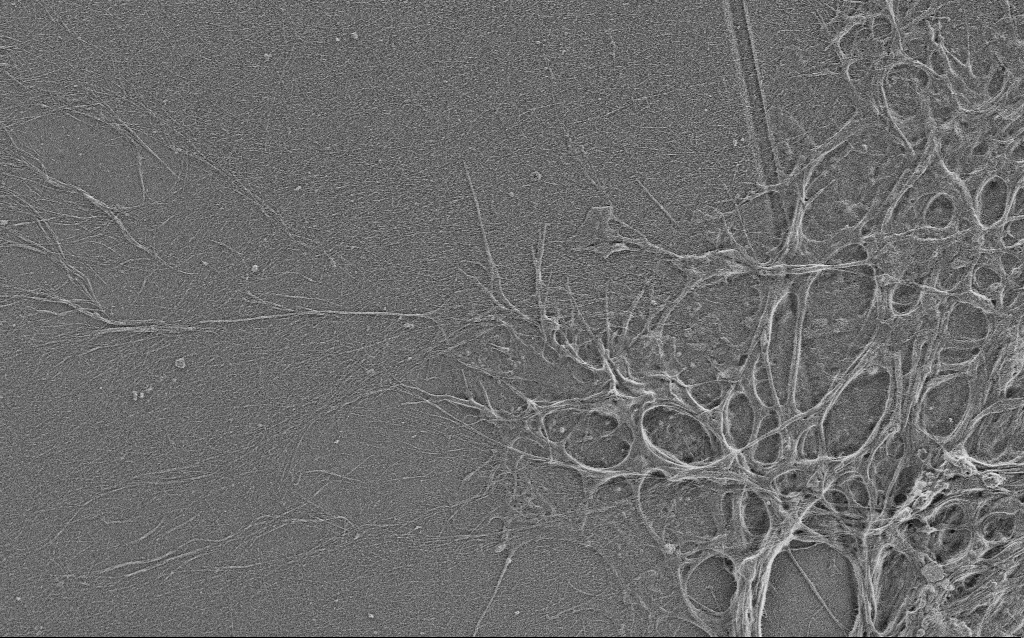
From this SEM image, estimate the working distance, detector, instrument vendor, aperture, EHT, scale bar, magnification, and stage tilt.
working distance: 4 mm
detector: SE2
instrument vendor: Zeiss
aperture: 30 µm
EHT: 0.9 kV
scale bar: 10000 nm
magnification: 5 K X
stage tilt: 0°